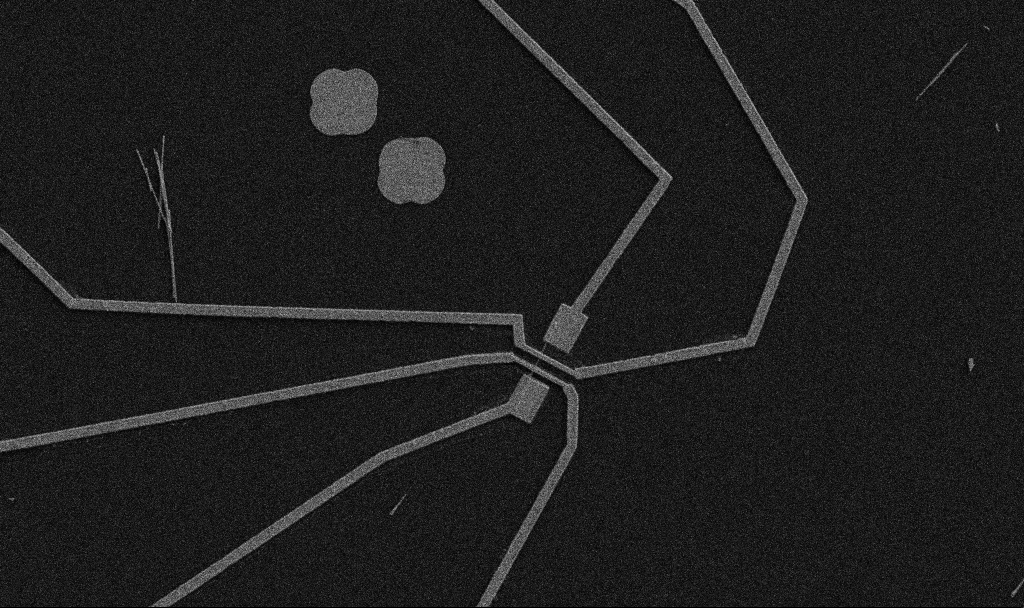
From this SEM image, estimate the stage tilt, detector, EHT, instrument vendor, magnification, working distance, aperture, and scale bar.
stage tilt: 0°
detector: SE2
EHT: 5 kV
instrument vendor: Zeiss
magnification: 5 K X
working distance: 10.7 mm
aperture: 30 µm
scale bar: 10000 nm